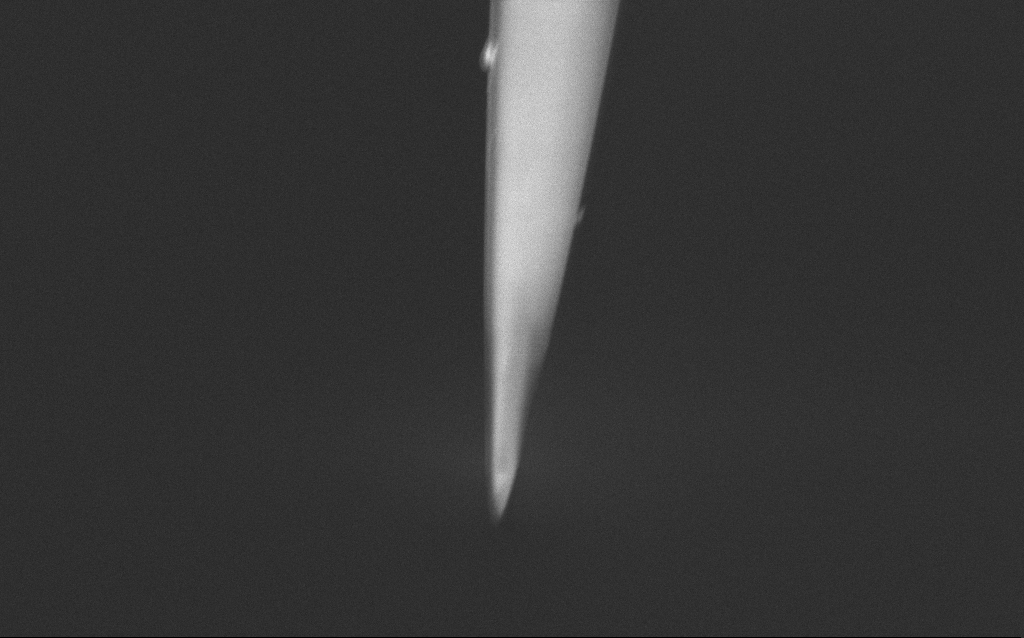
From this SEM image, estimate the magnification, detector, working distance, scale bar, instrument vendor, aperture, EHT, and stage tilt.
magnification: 14.31 K X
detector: InLens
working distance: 5 mm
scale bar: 1000 nm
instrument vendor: Zeiss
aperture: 30 µm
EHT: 1 kV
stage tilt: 45°